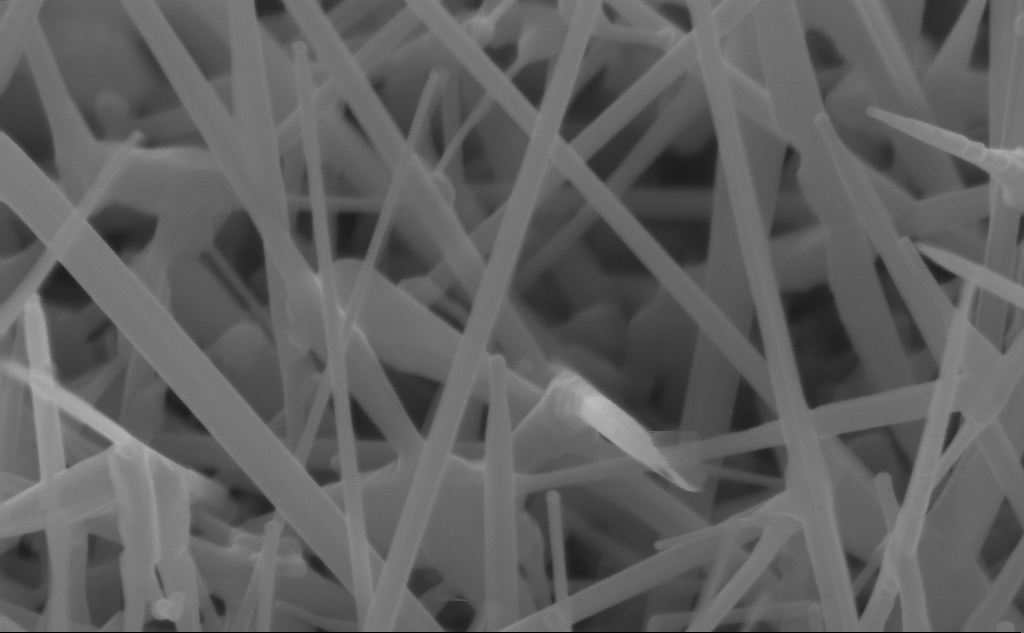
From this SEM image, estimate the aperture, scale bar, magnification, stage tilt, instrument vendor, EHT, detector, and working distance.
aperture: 30 µm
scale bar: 200 nm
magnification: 150 K X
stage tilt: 45°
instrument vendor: Zeiss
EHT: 10 kV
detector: InLens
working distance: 4 mm